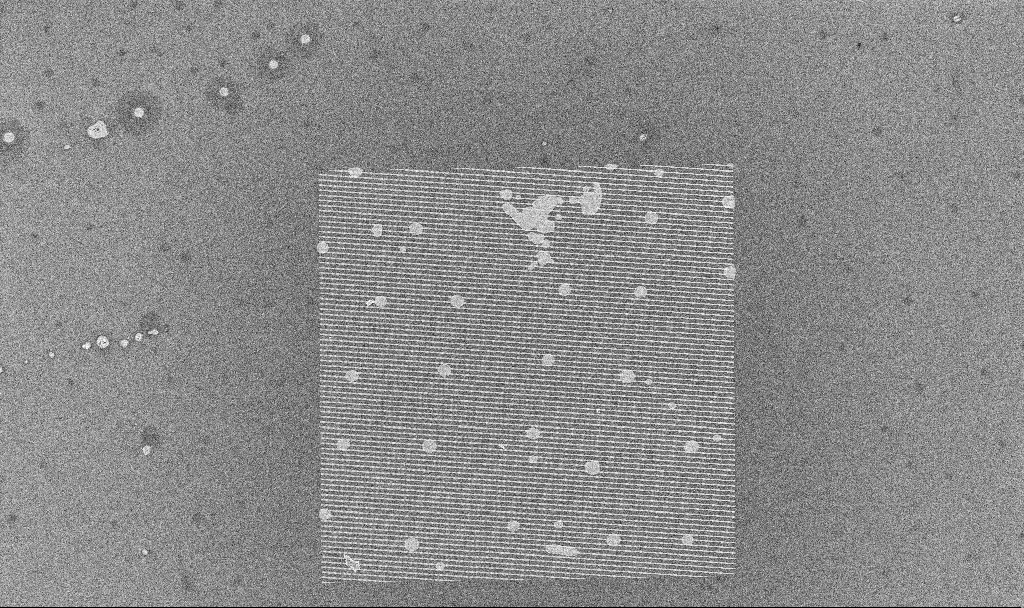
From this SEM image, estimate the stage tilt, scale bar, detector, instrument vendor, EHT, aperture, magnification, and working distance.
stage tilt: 0°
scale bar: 20000 nm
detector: InLens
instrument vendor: Zeiss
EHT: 5 kV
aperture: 30 µm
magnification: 1.53 K X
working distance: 4.6 mm